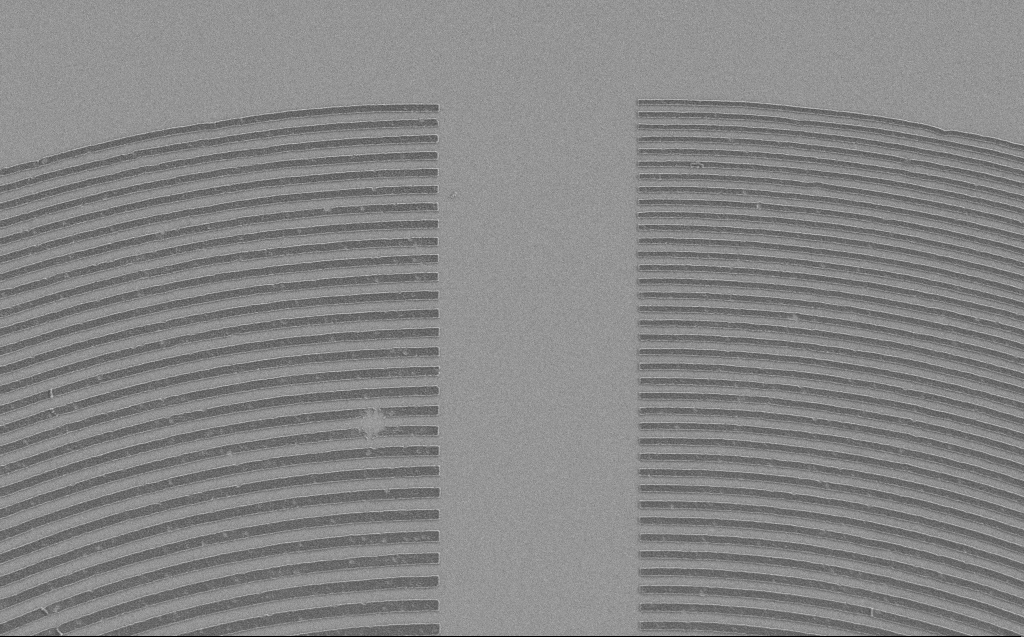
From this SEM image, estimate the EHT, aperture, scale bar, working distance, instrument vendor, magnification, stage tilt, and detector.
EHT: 1.2 kV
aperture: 30 µm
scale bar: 2000 nm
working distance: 5 mm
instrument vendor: Zeiss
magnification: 7.48 K X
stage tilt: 0°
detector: SE2